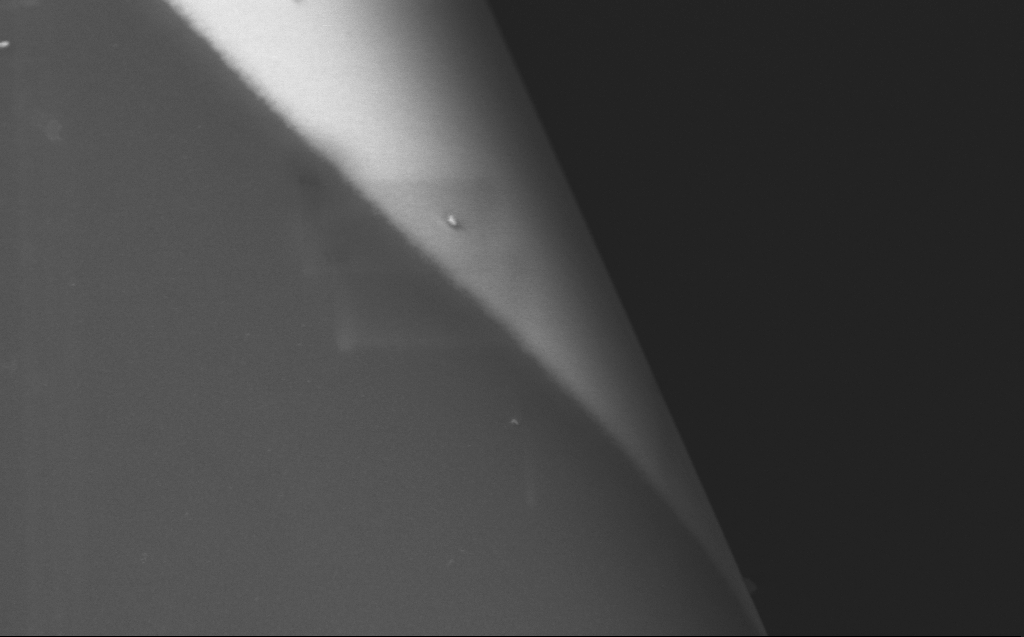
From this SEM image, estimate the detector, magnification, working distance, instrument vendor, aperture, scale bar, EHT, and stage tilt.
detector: InLens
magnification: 64.27 K X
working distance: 4 mm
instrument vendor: Zeiss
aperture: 30 µm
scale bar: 1000 nm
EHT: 5 kV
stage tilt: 45°